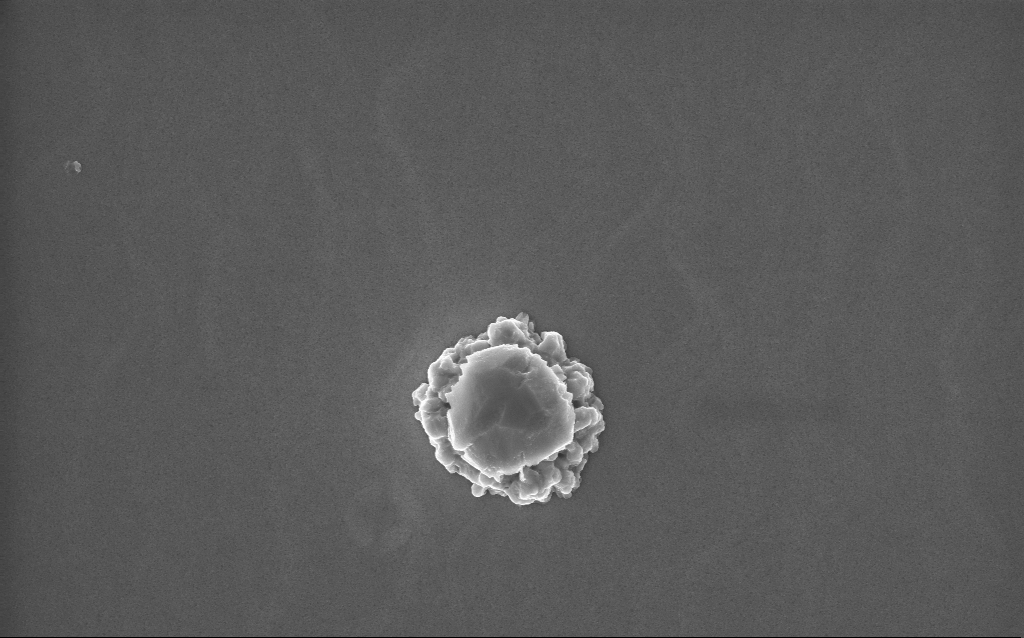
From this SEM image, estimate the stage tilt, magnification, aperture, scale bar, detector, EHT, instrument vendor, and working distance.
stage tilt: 0°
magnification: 38.48 K X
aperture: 30 µm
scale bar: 1000 nm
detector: InLens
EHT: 10 kV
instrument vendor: Zeiss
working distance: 2 mm